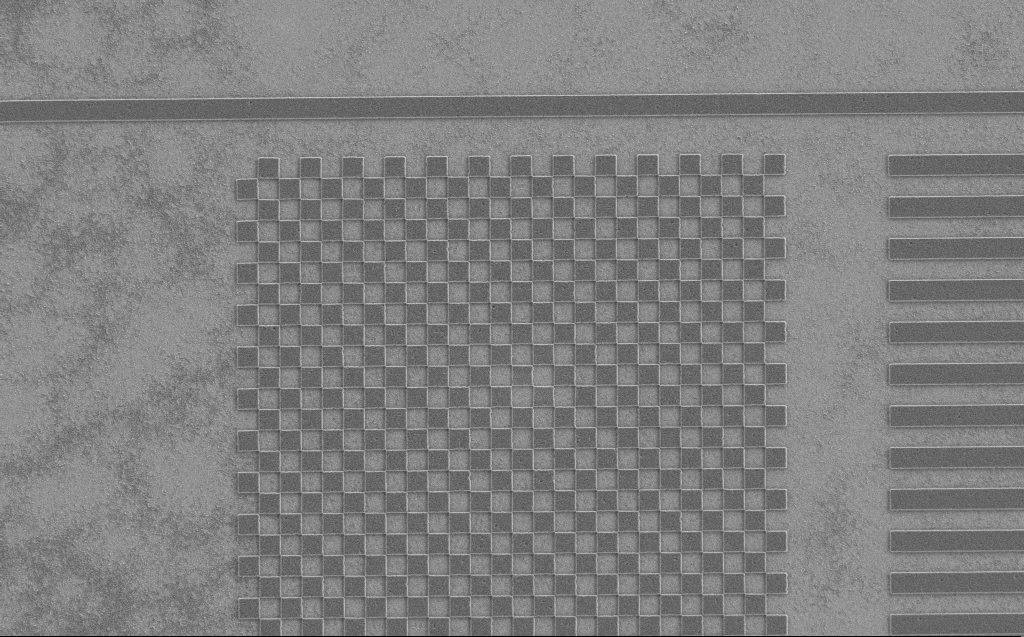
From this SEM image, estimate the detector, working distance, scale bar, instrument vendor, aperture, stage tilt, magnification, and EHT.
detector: SE2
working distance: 5 mm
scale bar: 2000 nm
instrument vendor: Zeiss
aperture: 30 µm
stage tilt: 0°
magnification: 7.75 K X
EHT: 1.2 kV